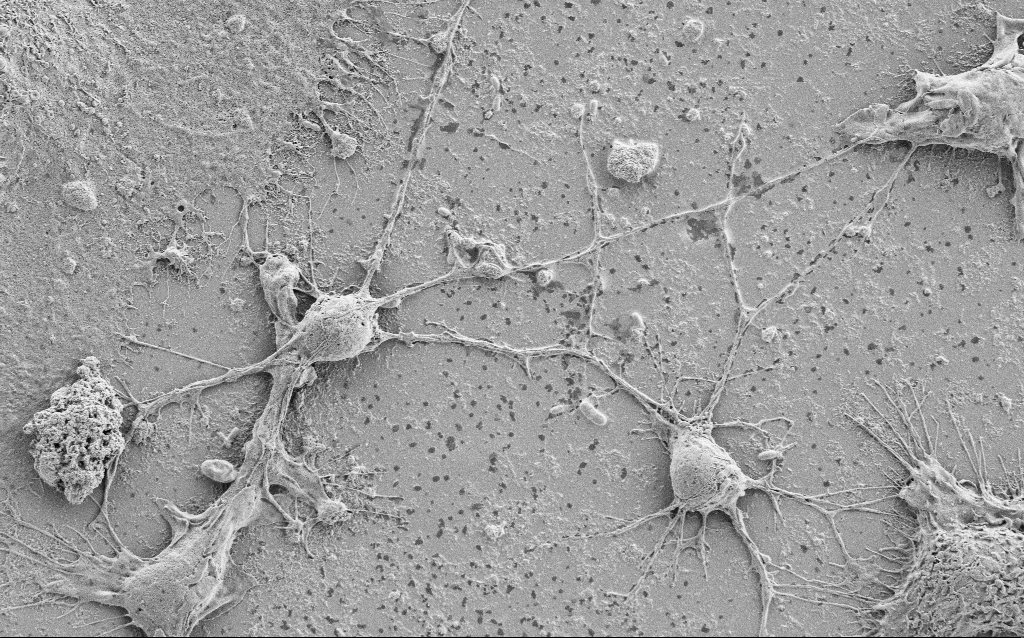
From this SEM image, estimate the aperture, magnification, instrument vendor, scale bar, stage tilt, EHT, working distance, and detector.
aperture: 30 µm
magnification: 3.5 K X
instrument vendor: Zeiss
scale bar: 10000 nm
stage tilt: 0°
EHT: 1.5 kV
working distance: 6.8 mm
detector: SE2